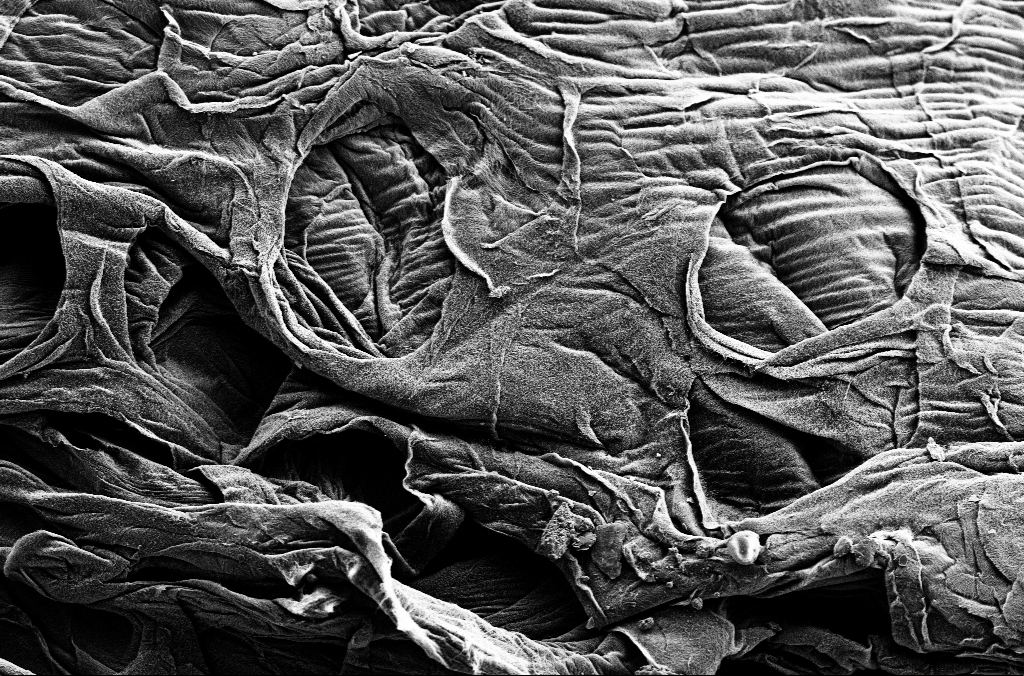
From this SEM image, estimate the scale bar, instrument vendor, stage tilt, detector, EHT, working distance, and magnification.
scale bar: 10000 nm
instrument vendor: Zeiss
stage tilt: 0°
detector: SE2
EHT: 1.8 kV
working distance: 5.4 mm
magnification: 2.5 K X